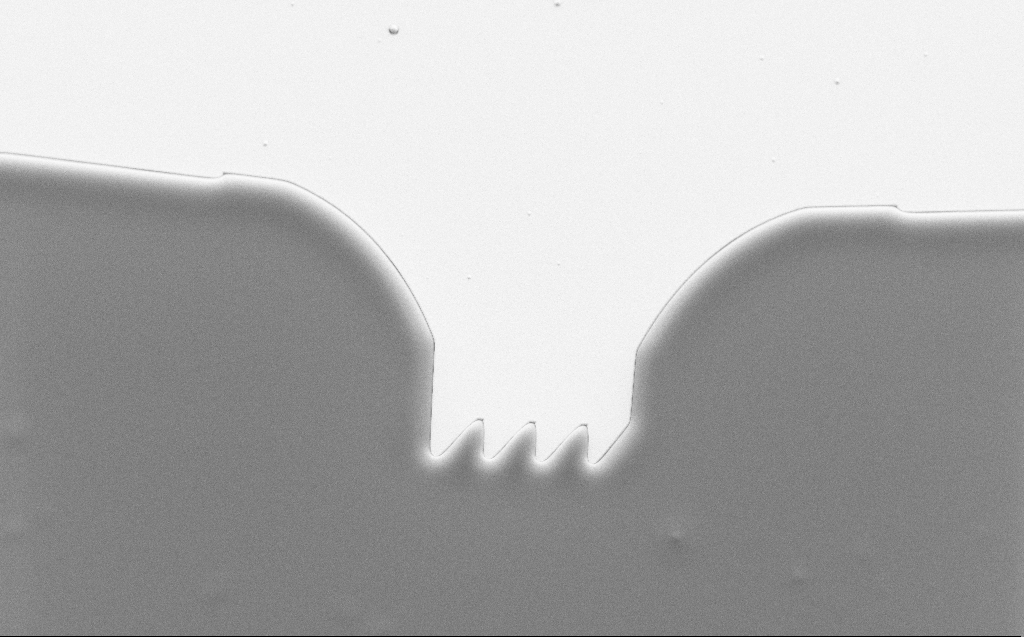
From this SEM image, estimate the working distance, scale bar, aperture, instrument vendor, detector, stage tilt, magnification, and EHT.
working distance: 6 mm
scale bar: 20000 nm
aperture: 30 µm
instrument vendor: Zeiss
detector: SE2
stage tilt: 30°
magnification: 1.67 K X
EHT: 1.1 kV